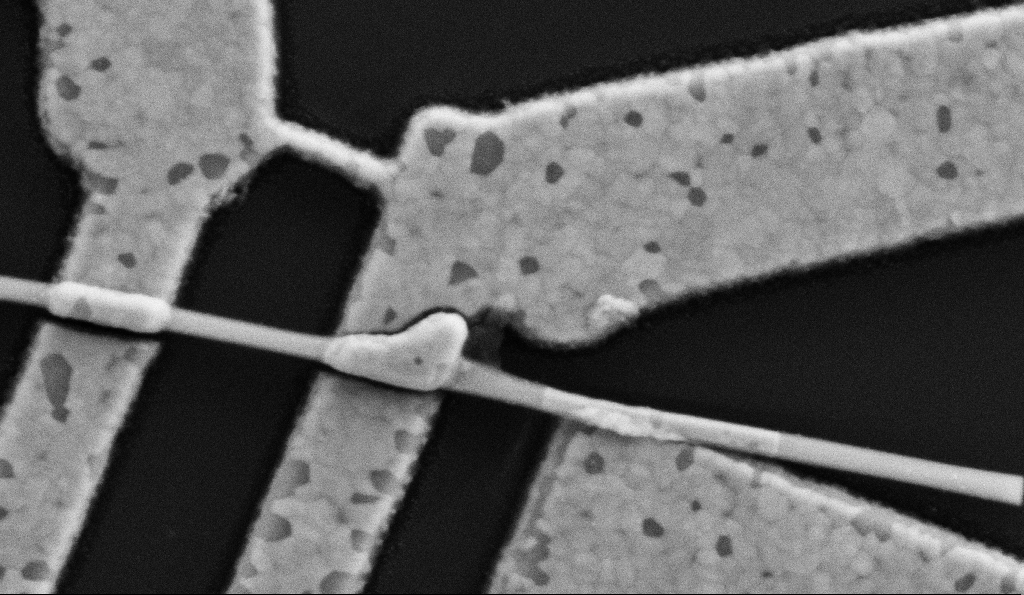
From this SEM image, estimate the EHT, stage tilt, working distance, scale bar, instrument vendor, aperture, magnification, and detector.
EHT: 5 kV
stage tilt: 0°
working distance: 8.5 mm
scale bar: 200 nm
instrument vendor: Zeiss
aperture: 30 µm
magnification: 100 K X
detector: SE2